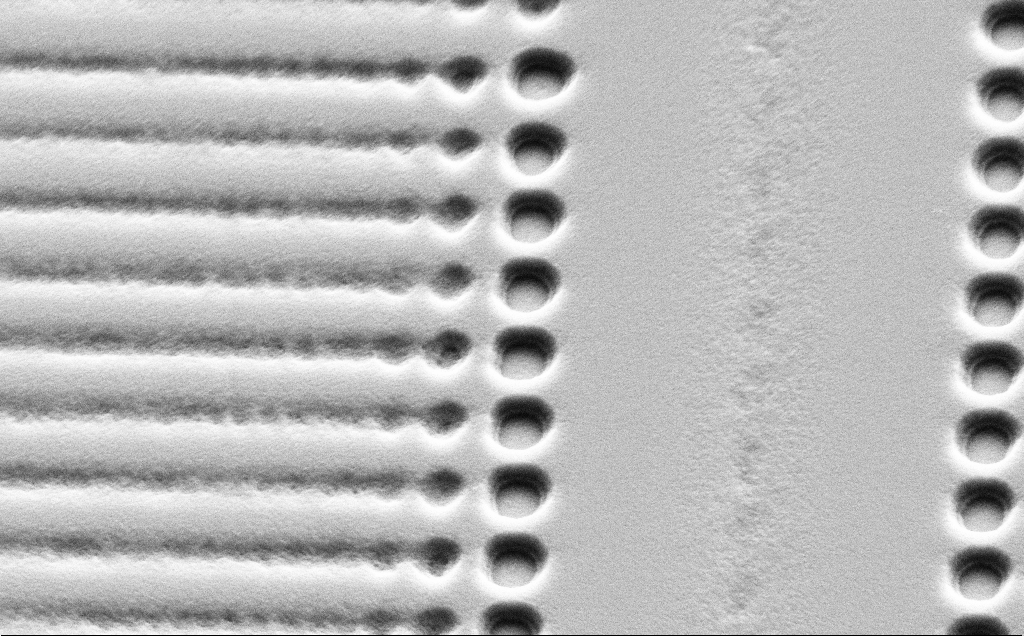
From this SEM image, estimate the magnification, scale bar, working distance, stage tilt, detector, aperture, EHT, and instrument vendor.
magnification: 12.86 K X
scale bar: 2000 nm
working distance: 10 mm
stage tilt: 45°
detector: SE2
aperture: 30 µm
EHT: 5 kV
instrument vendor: Zeiss